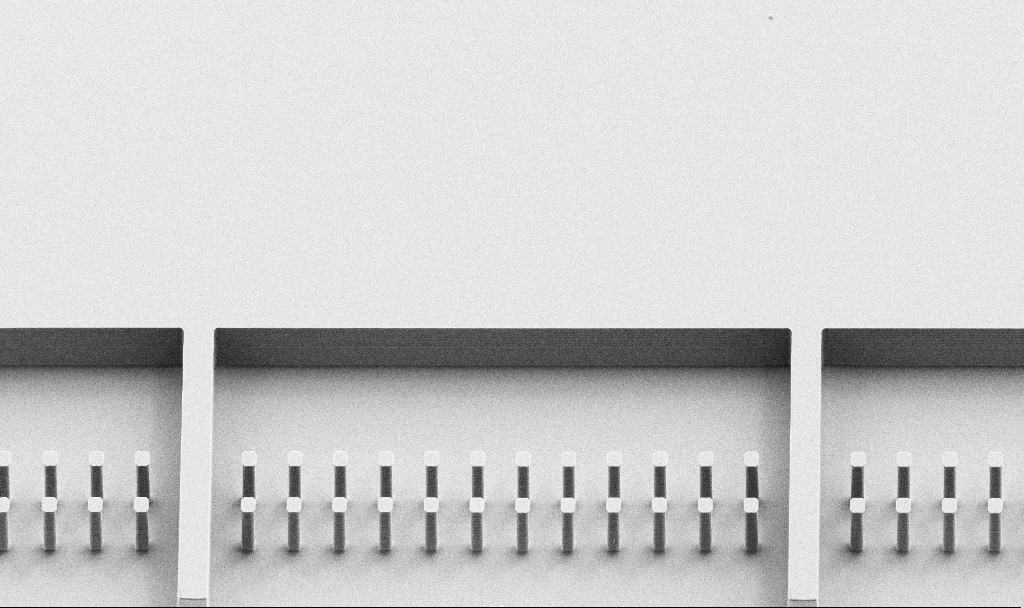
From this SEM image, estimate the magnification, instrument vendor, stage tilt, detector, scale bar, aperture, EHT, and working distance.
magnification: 0.564 K X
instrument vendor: Zeiss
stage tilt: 45°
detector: SE2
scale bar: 100000 nm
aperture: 30 µm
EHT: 10 kV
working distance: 6.6 mm